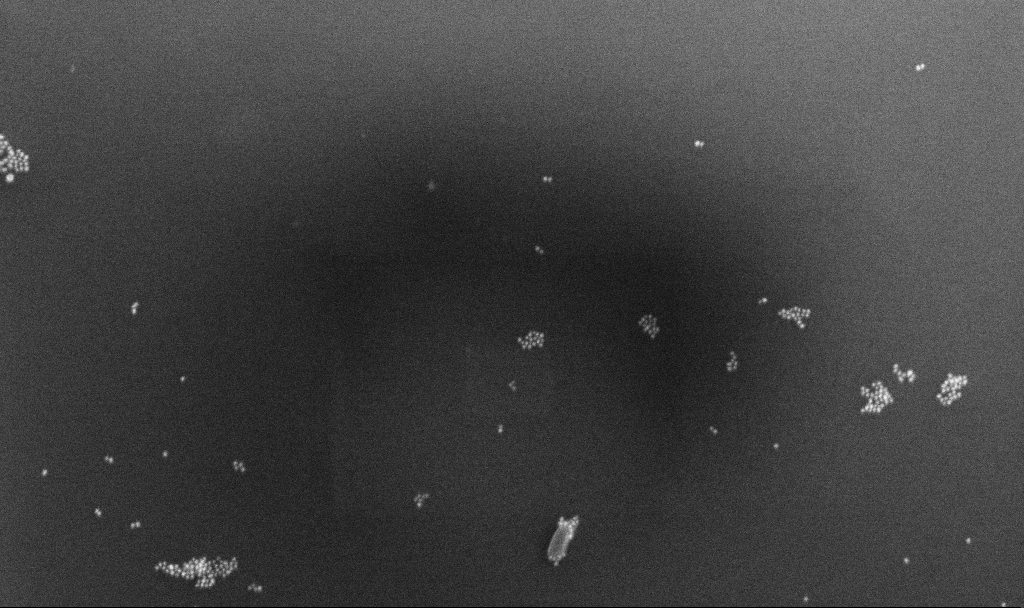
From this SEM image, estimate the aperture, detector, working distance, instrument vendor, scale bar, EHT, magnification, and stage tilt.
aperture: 30 µm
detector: InLens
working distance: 4.3 mm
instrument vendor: Zeiss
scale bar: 200 nm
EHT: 10 kV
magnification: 100 K X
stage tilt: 0°